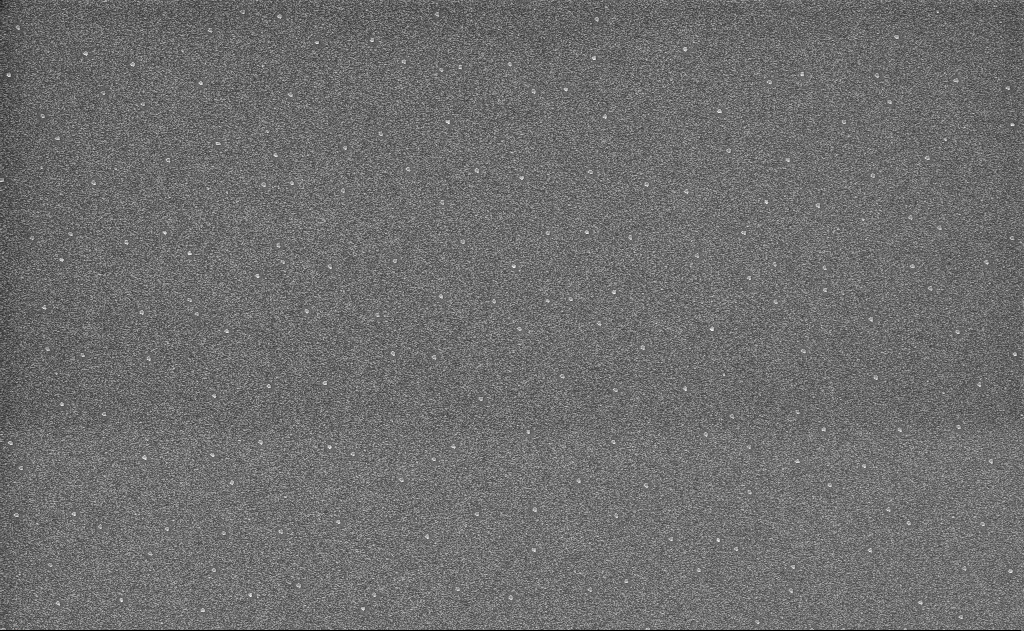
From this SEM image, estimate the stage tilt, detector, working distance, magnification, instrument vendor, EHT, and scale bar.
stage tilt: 0°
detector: InLens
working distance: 15 mm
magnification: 10 K X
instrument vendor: Zeiss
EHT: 10 kV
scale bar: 2000 nm